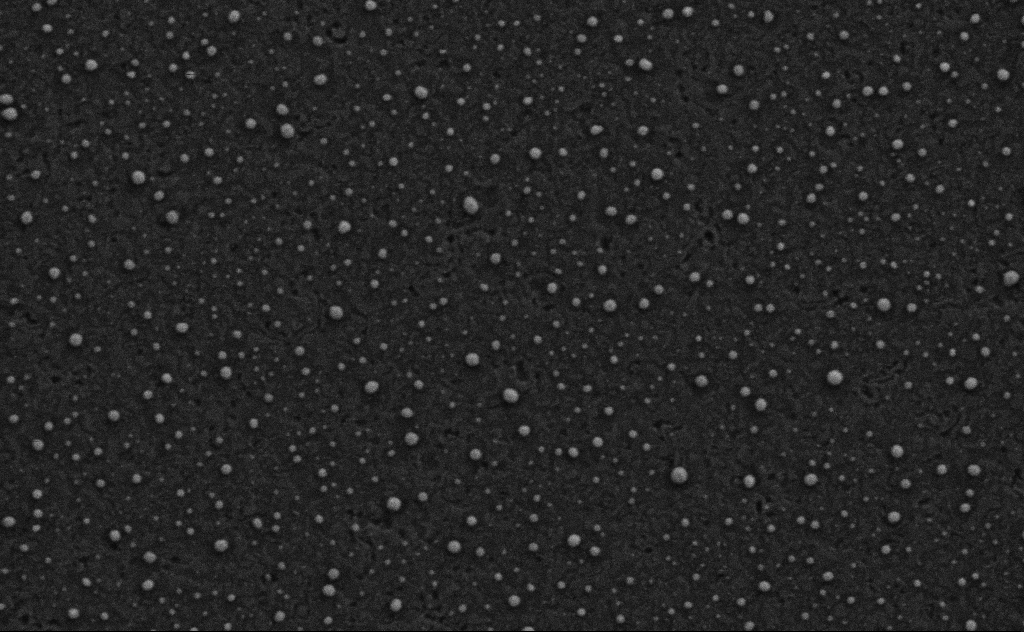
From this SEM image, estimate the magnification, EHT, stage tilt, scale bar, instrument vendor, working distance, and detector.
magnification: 80 K X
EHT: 3 kV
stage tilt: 0°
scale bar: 200 nm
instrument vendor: Zeiss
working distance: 4 mm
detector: SE2